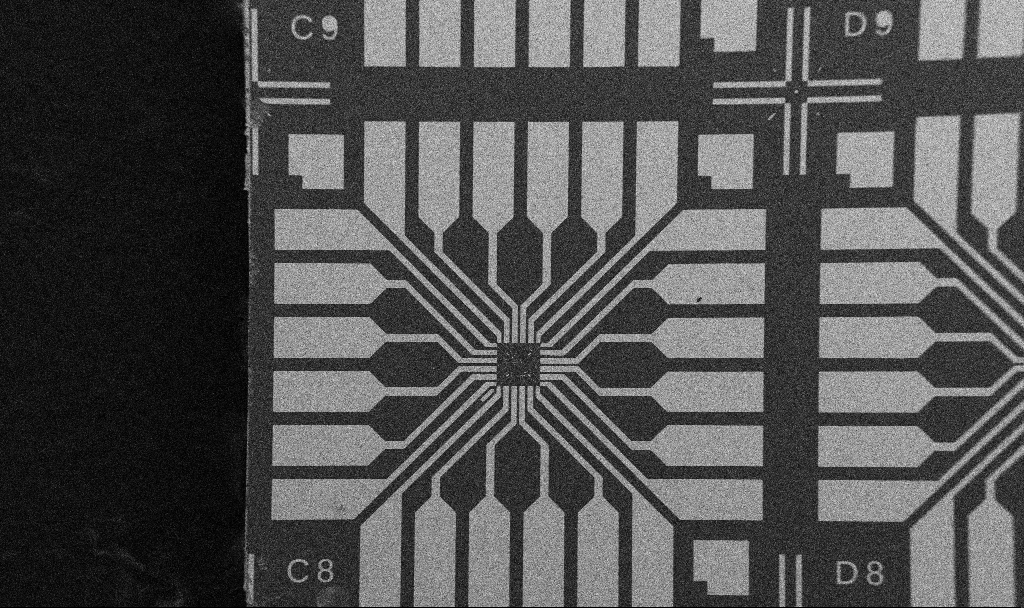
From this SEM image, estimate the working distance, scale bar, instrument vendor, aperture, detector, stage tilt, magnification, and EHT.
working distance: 10.7 mm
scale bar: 200000 nm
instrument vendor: Zeiss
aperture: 30 µm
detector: SE2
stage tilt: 0°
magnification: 0.1 K X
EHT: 5 kV